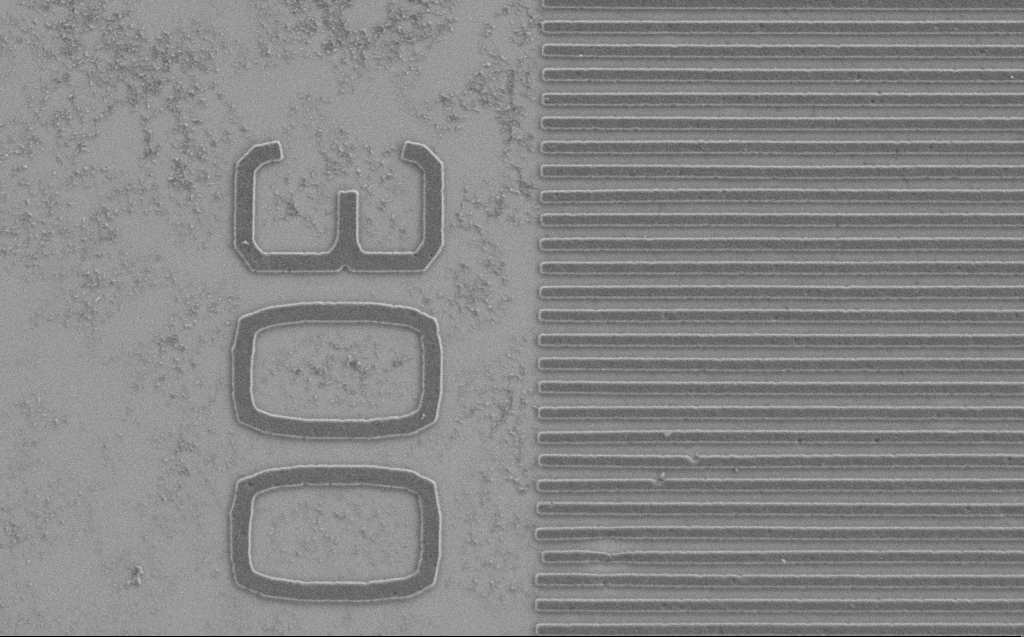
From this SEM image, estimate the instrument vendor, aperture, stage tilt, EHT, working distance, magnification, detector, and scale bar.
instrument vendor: Zeiss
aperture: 30 µm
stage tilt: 0°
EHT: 1.2 kV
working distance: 5 mm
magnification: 14.86 K X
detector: SE2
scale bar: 1000 nm